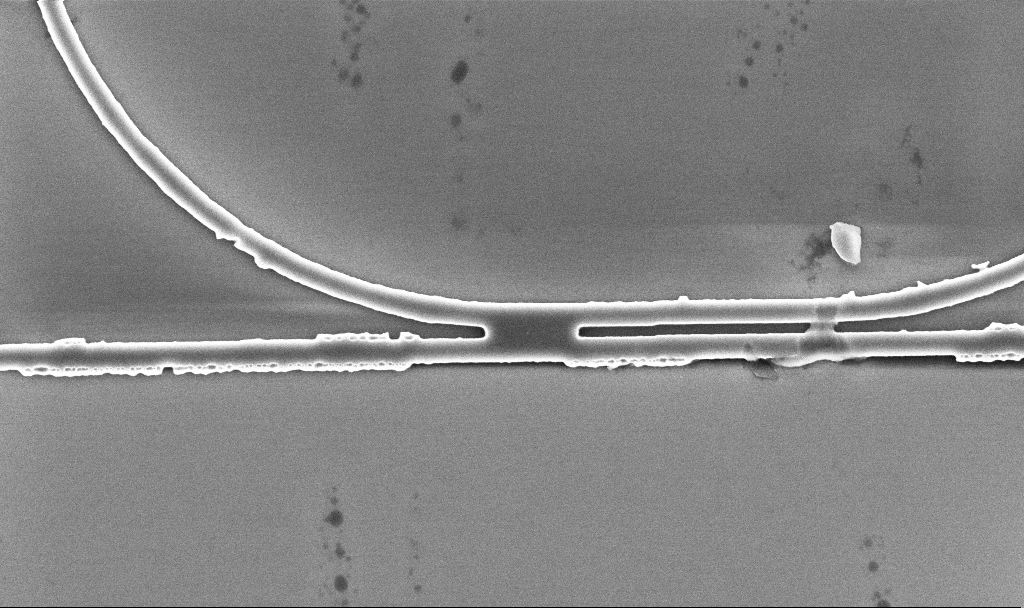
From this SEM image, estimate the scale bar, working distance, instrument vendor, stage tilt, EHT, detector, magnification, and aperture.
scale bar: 2000 nm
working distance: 5.2 mm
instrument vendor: Zeiss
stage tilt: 0°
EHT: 5 kV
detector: InLens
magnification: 18.76 K X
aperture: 30 µm